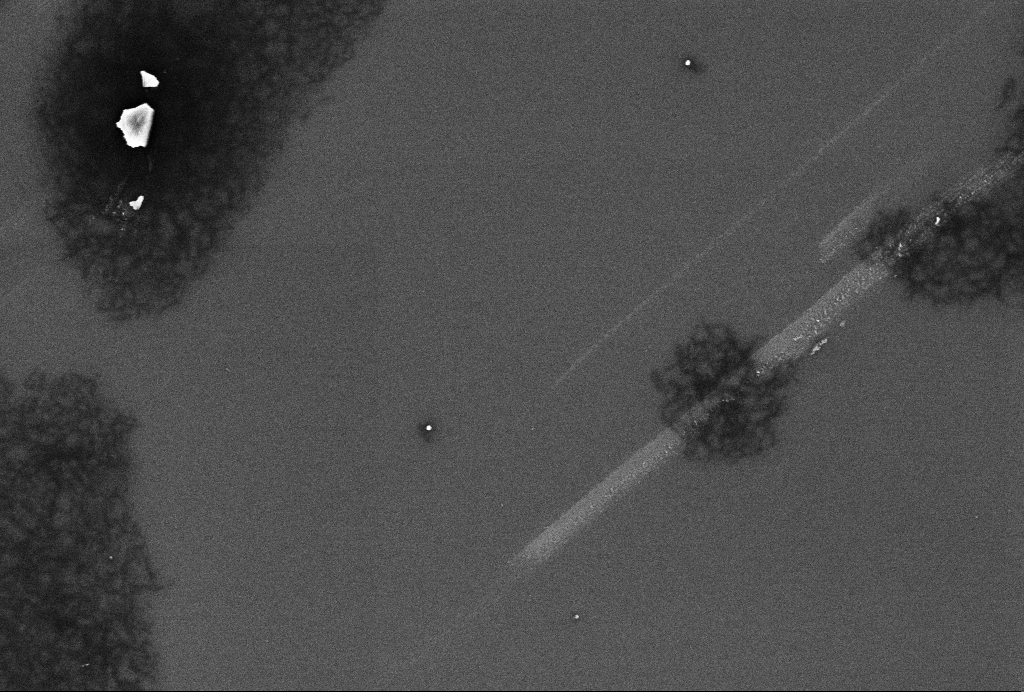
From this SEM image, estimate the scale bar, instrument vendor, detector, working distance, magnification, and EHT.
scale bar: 200 nm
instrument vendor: Zeiss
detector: InLens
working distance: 3.3 mm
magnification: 68.88 K X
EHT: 2 kV